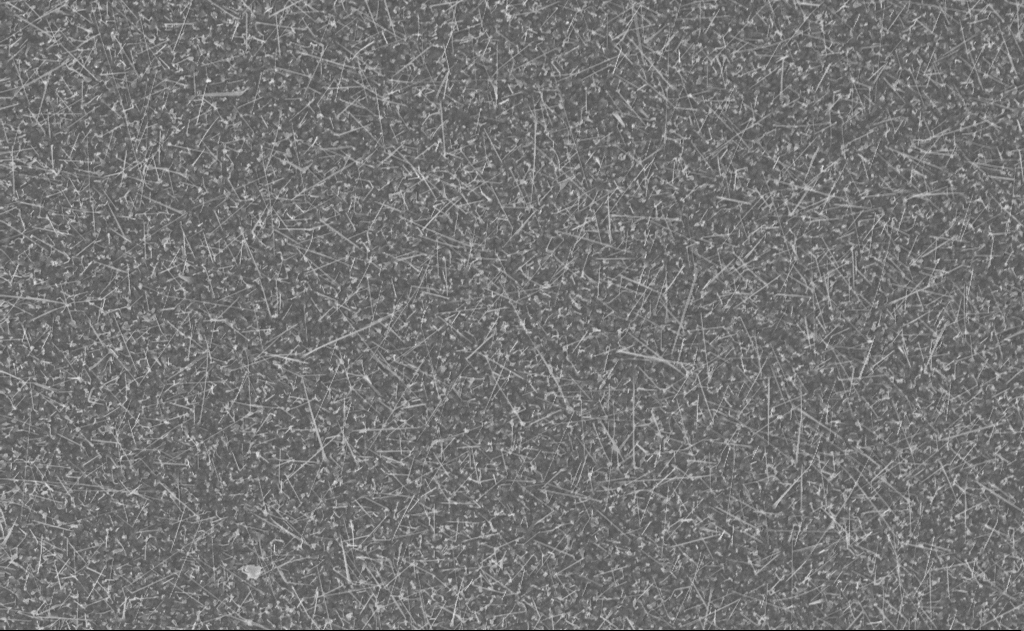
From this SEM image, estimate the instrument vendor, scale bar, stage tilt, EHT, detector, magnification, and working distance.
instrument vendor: Zeiss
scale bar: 2000 nm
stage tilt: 0°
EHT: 10 kV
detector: InLens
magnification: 10 K X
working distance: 20 mm